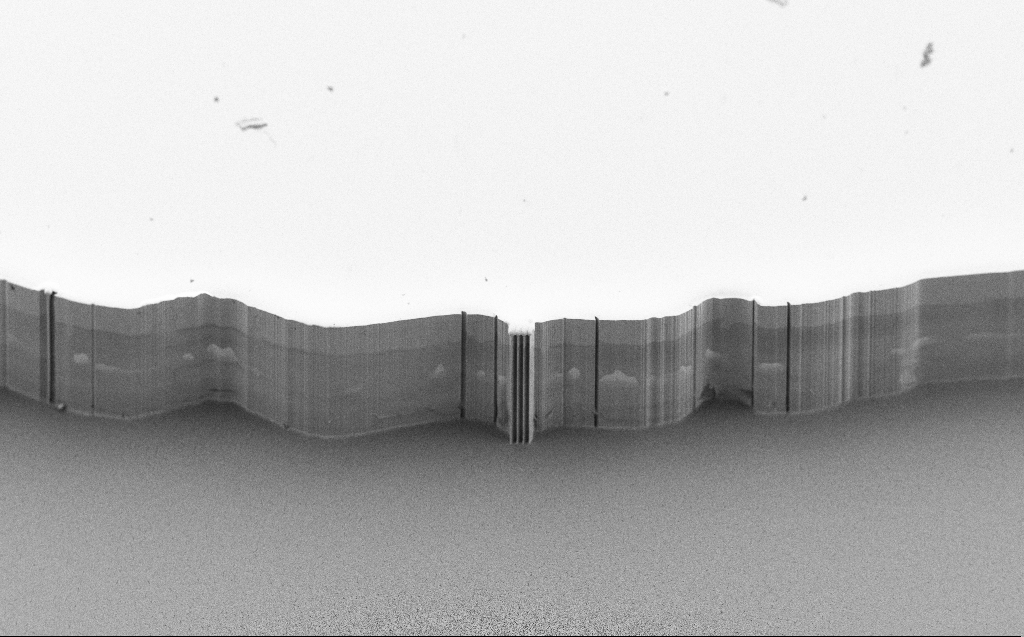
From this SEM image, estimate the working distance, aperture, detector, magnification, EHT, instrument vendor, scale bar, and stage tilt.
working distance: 5 mm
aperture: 30 µm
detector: SE2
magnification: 0.197 K X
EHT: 5 kV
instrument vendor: Zeiss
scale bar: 100000 nm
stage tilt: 45°